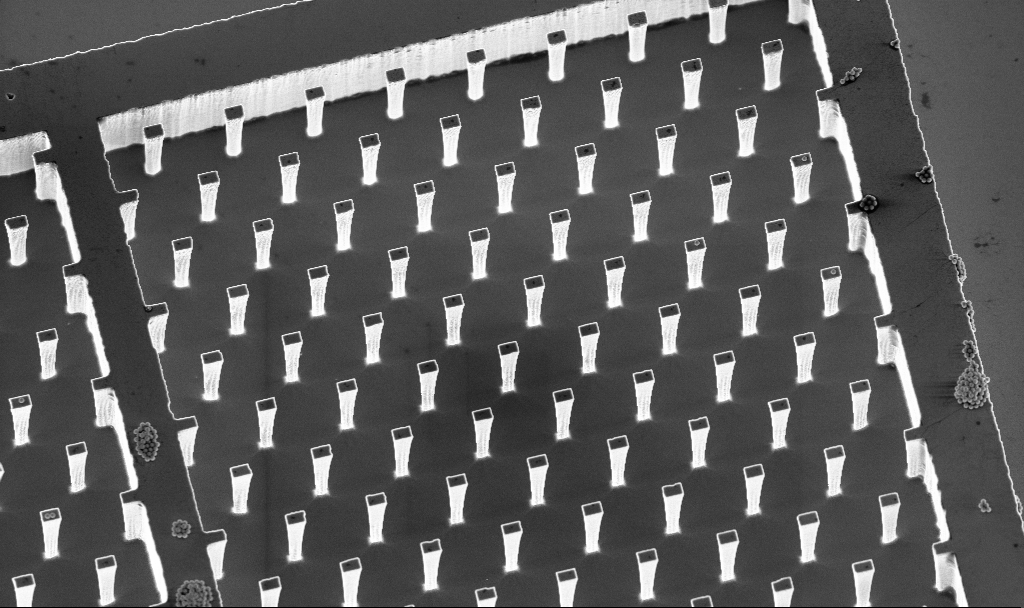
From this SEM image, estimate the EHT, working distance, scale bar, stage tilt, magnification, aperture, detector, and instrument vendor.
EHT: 5 kV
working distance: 4.7 mm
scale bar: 10000 nm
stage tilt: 20°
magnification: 2.55 K X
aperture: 30 µm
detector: InLens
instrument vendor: Zeiss